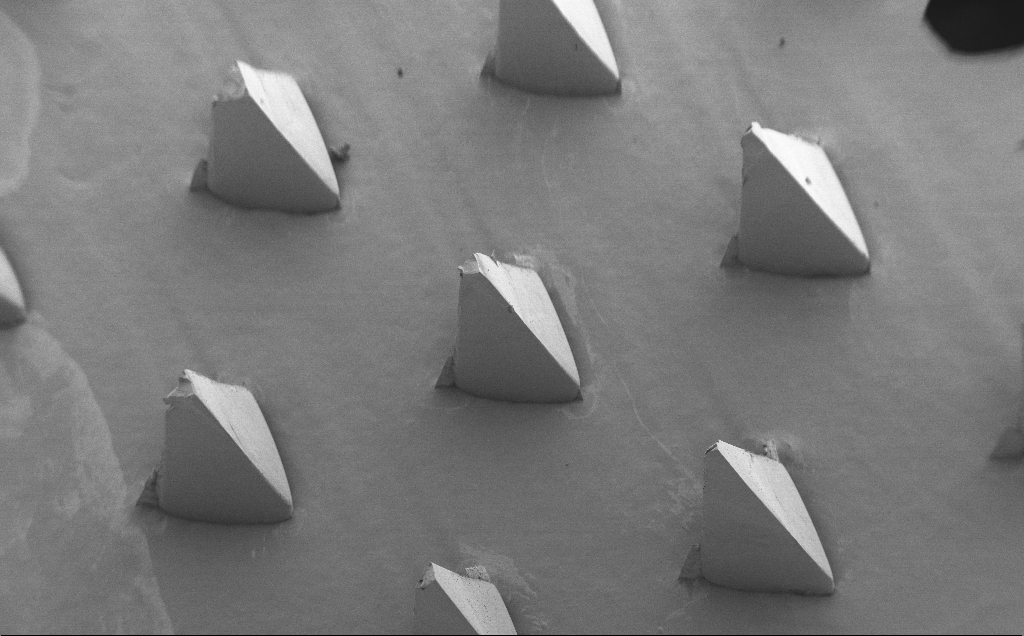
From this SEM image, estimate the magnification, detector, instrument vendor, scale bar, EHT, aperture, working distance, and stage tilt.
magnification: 0.077 K X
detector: SE2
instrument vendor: Zeiss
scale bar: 200000 nm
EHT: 5 kV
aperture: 30 µm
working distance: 8 mm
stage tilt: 40°